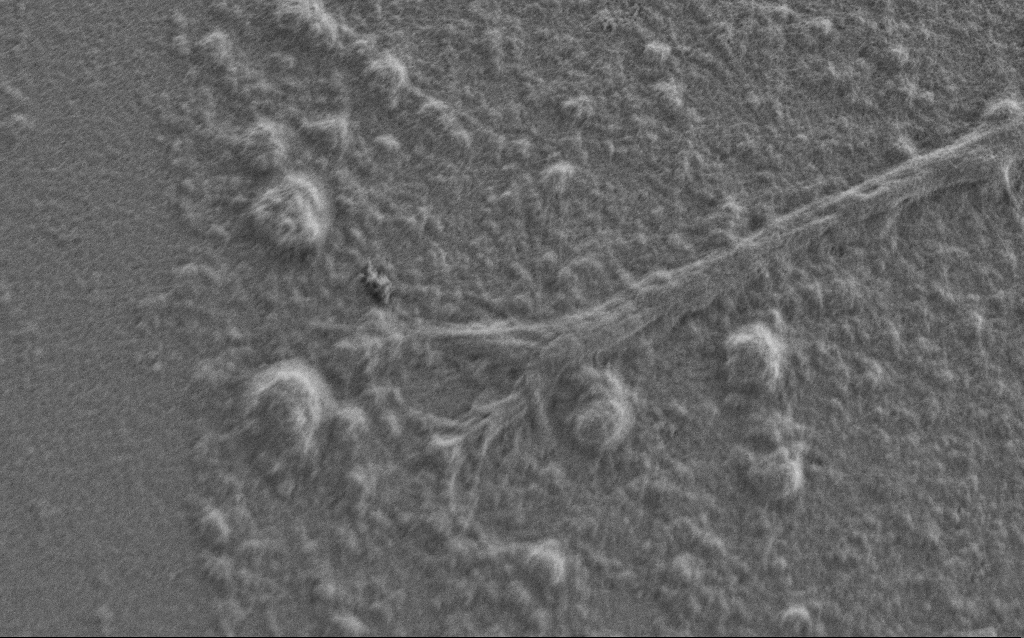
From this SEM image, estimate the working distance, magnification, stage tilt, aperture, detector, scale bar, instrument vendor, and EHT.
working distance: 7 mm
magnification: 7.5 K X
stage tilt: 0°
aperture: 30 µm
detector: SE2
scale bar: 2000 nm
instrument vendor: Zeiss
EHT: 0.9 kV